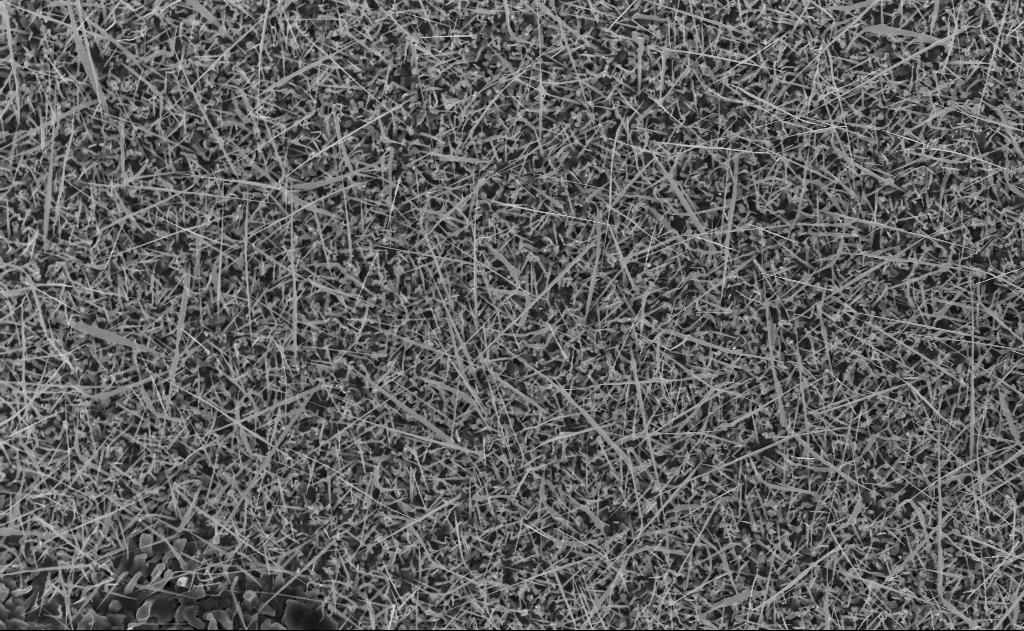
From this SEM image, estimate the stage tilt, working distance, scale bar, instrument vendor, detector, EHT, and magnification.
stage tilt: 0°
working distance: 10 mm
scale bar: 2000 nm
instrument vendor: Zeiss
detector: InLens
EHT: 10 kV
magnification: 10 K X